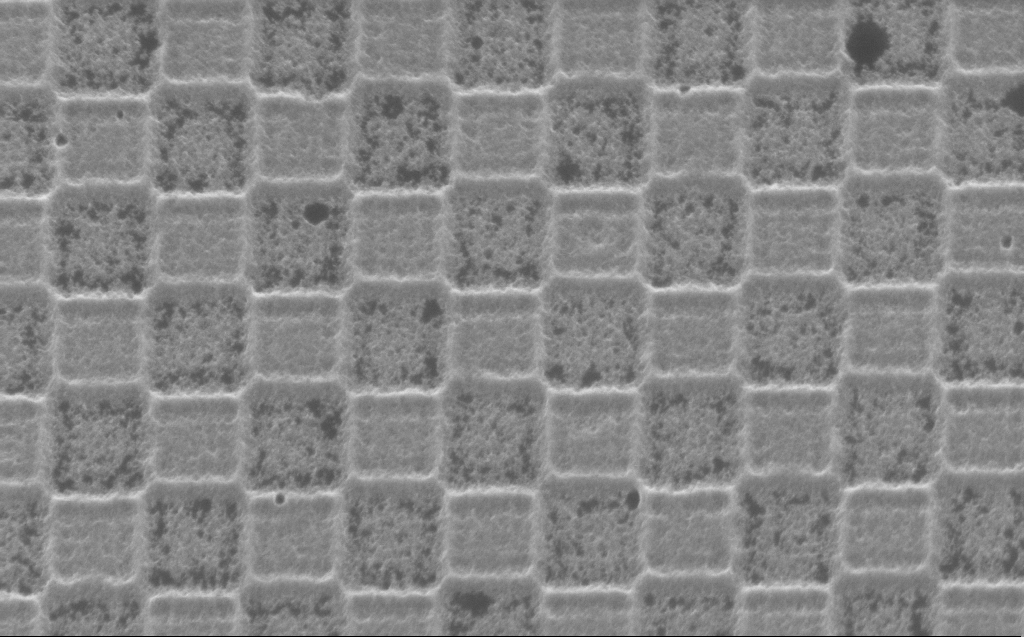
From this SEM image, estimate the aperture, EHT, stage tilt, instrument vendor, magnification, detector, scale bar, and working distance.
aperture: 30 µm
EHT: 5 kV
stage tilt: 30°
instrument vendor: Zeiss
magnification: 73.63 K X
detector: InLens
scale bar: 200 nm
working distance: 4 mm